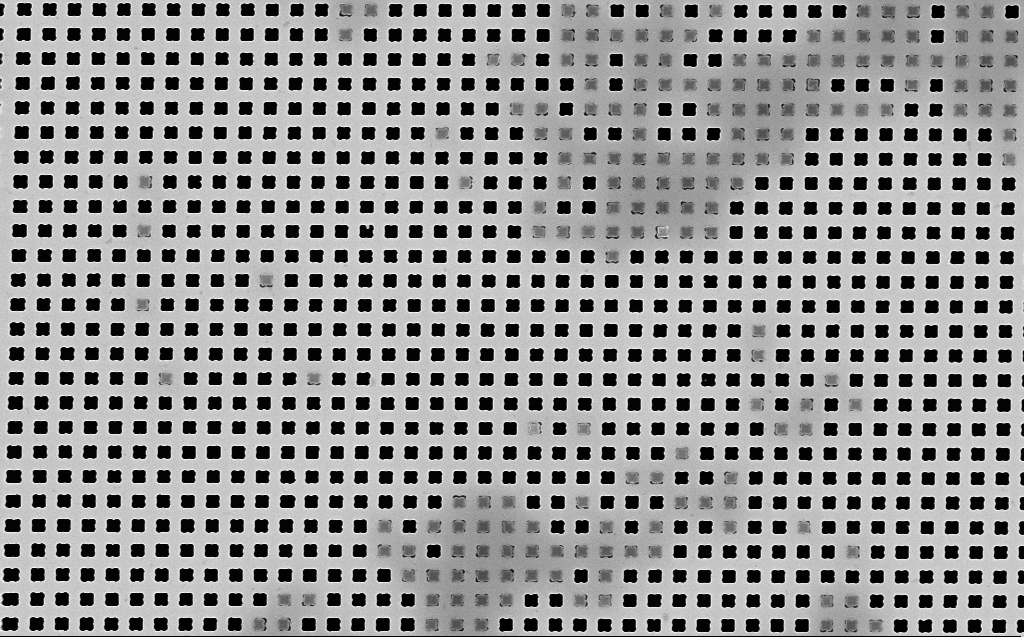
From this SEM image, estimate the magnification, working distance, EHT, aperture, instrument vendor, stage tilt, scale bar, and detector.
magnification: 18.31 K X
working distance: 7 mm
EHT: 10 kV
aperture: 30 µm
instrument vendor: Zeiss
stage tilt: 0°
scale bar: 1000 nm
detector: InLens